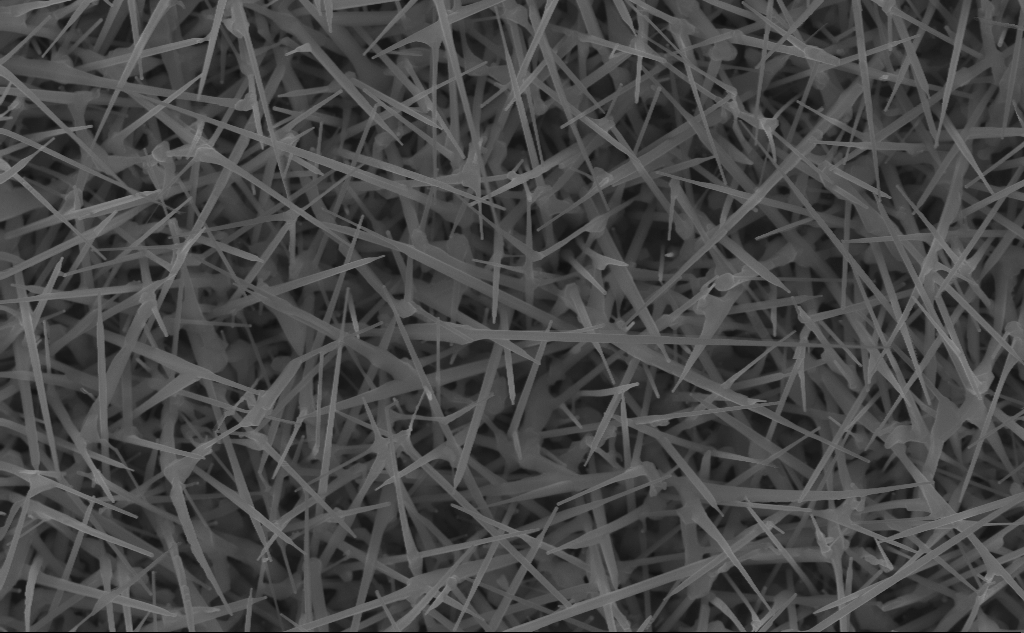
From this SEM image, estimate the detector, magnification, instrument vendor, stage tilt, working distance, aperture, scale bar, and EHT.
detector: InLens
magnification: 40 K X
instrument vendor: Zeiss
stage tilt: -0°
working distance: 7 mm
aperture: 30 µm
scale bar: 1000 nm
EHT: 10 kV